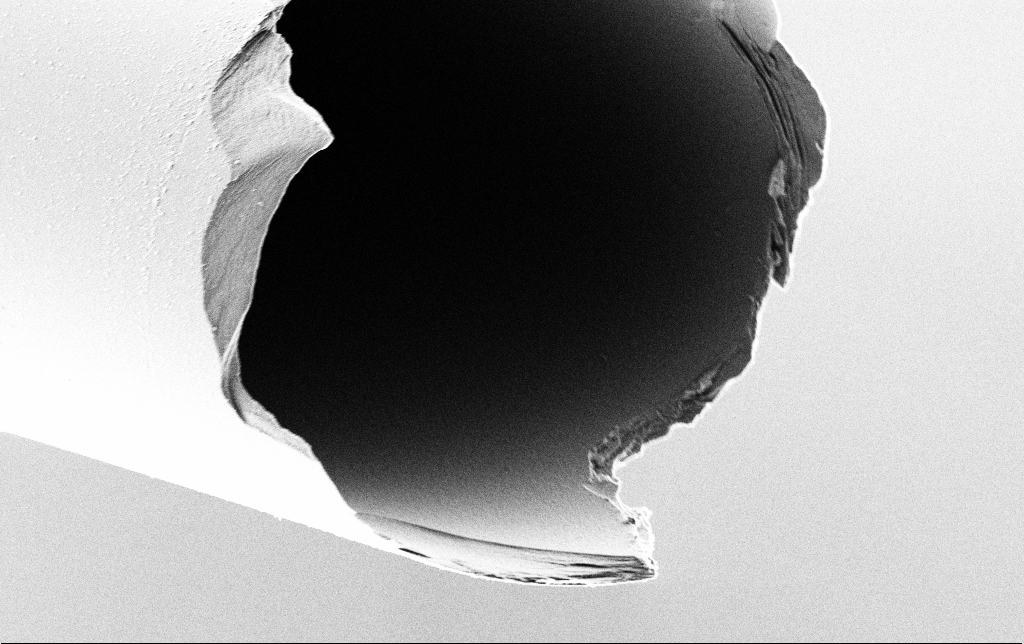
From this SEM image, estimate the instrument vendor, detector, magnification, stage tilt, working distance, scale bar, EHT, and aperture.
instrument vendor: Zeiss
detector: SE2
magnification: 5 K X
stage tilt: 45°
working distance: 7.2 mm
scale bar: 10000 nm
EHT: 2 kV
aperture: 30 µm